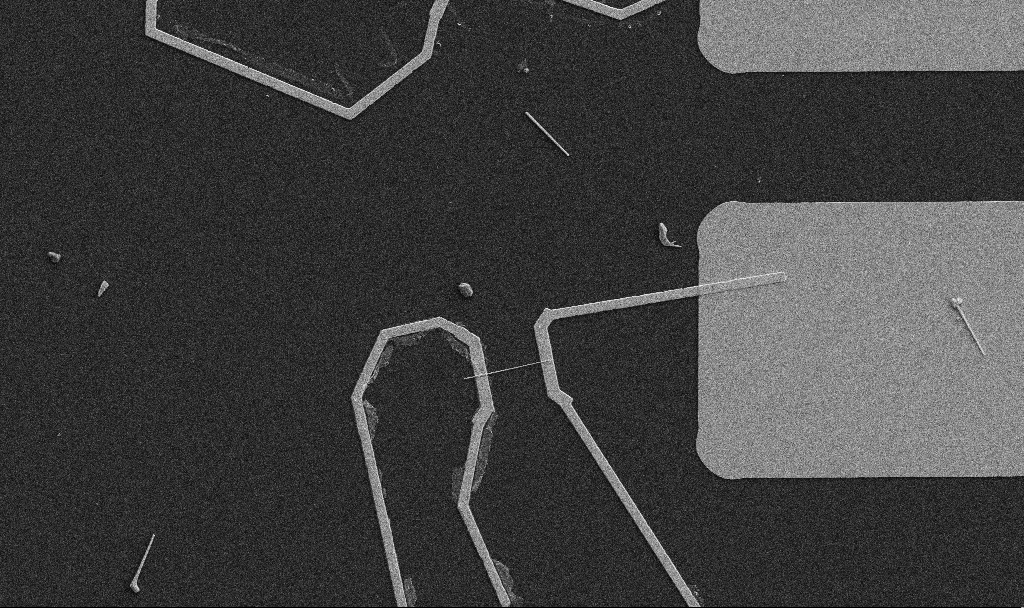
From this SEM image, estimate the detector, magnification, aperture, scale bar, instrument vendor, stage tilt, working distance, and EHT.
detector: SE2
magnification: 5 K X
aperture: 30 µm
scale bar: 10000 nm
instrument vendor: Zeiss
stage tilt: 0°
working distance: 10.7 mm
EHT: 5 kV